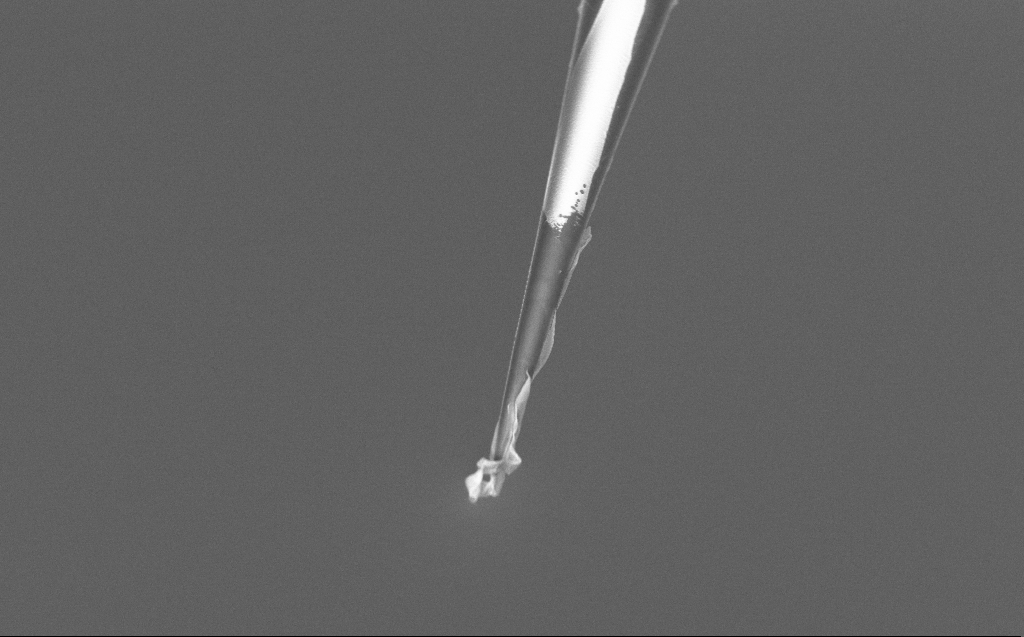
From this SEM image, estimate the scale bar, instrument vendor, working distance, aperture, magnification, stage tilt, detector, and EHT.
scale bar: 10000 nm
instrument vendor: Zeiss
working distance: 6 mm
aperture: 30 µm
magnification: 5 K X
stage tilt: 45°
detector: InLens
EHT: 5 kV